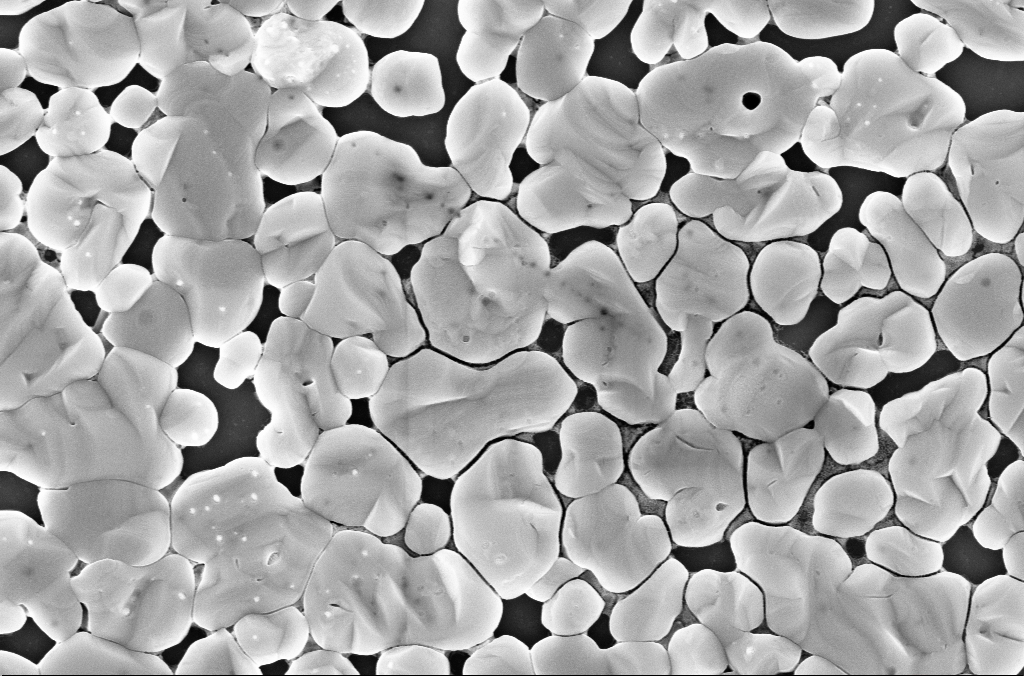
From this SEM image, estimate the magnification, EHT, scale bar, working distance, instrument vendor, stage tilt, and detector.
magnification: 49.38 K X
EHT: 5 kV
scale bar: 1000 nm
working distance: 3 mm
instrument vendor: Zeiss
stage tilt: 0°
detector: InLens